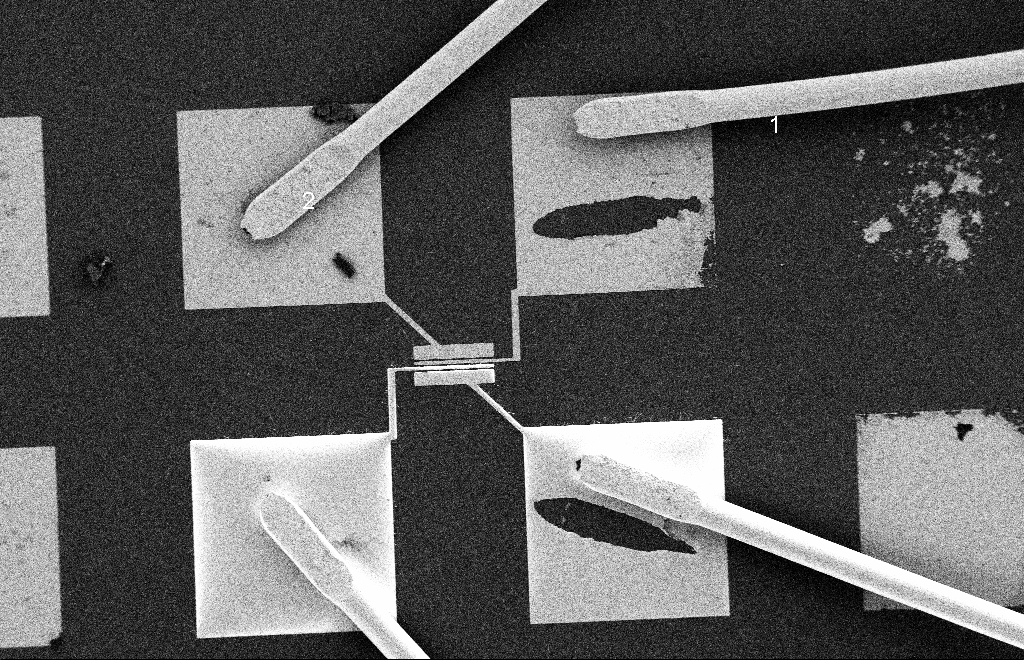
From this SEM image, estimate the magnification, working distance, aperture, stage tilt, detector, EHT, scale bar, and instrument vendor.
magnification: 0.481 K X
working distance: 9 mm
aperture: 20 µm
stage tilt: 0°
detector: SE2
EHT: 2 kV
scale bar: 20000 nm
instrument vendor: Zeiss